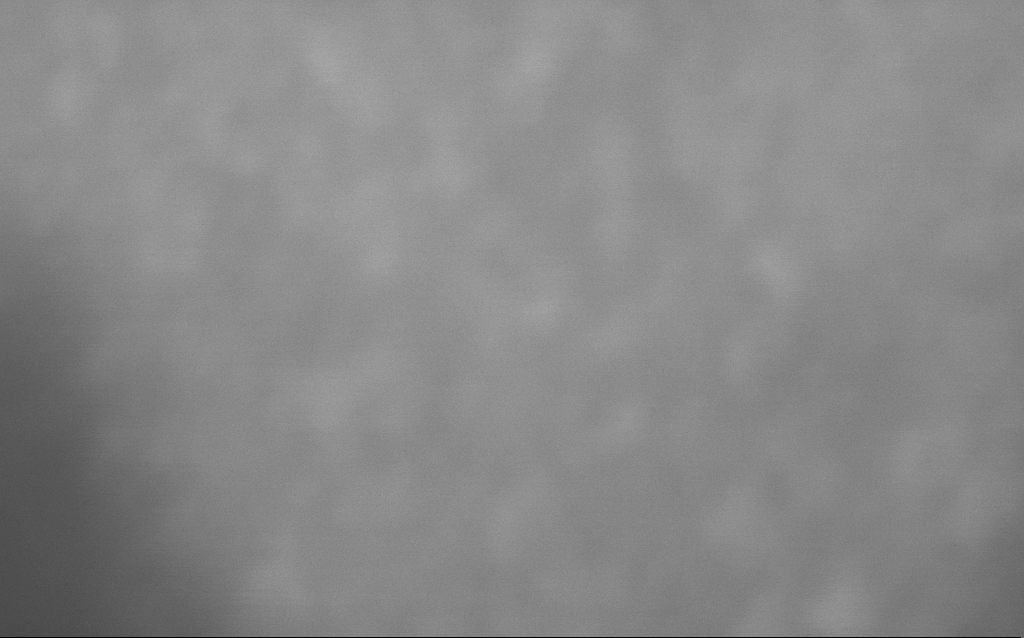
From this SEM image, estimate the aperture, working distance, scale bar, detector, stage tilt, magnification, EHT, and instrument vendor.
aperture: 30 µm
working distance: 3 mm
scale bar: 20 nm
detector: InLens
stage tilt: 0°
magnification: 3116.92 K X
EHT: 10 kV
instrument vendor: Zeiss